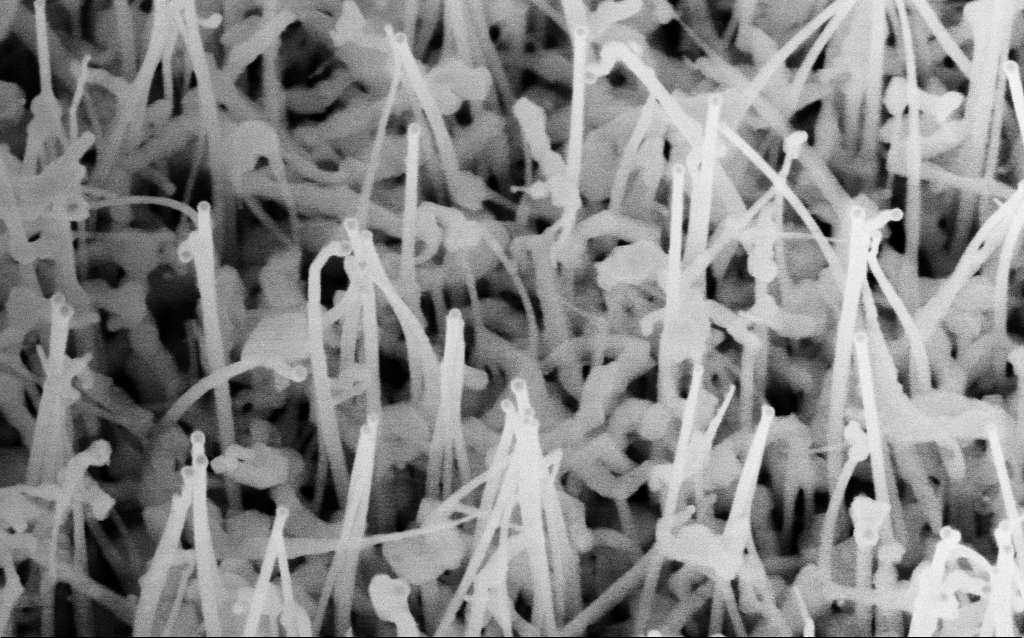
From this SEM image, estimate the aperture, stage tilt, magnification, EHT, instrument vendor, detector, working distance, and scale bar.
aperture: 30 µm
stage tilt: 35°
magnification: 149.8 K X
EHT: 10 kV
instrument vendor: Zeiss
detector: InLens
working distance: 7.3 mm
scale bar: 100 nm